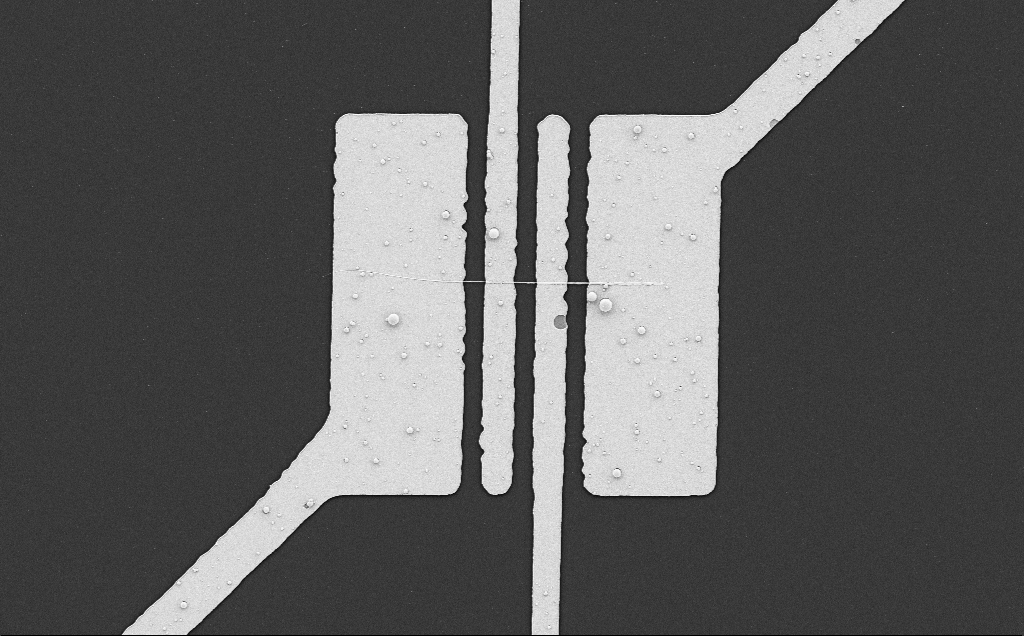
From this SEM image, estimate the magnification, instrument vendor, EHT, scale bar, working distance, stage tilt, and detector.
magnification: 4.69 K X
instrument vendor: Zeiss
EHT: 5 kV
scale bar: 10000 nm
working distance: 10 mm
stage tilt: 0°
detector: SE2